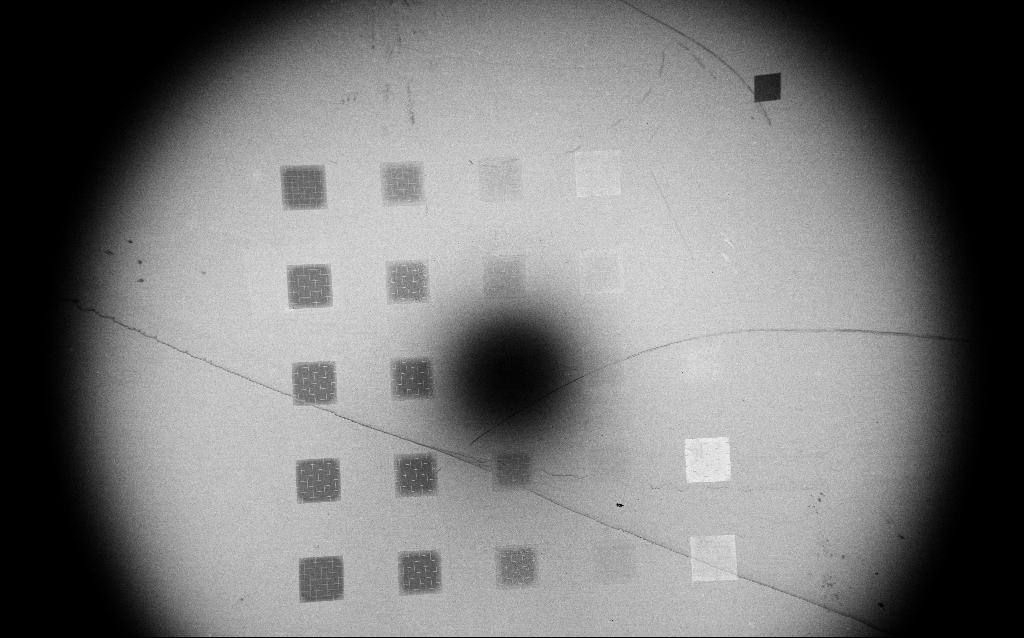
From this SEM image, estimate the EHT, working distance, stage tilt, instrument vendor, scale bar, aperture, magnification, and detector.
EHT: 3 kV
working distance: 2.7 mm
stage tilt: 0°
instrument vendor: Zeiss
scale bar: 200000 nm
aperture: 30 µm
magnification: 0.09 K X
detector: InLens